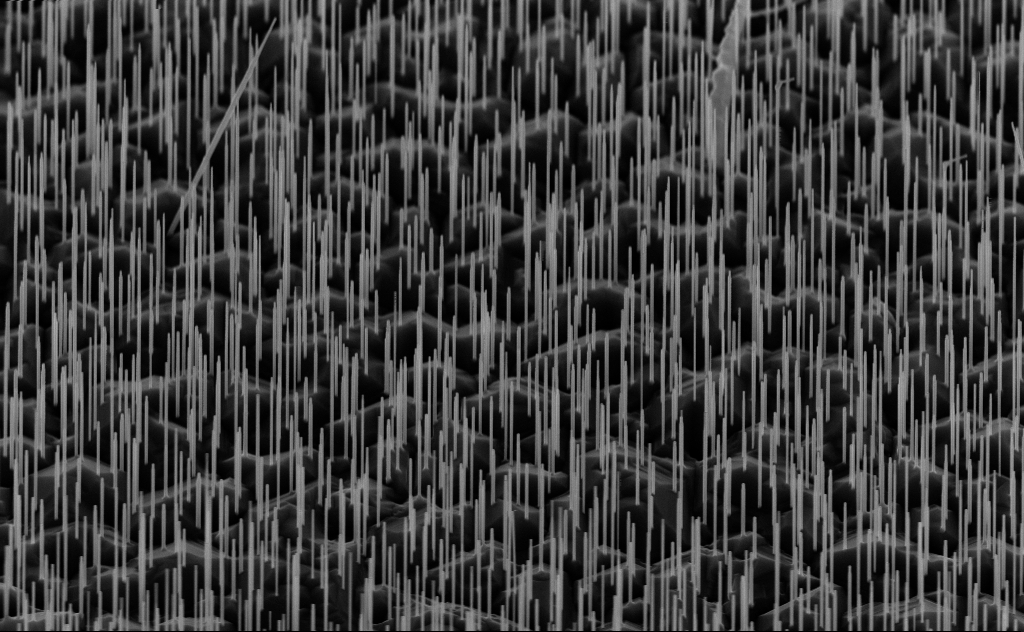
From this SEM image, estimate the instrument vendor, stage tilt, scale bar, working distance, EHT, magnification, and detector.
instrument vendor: Zeiss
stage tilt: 44.2°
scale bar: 2000 nm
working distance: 6 mm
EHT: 10 kV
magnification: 20 K X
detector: InLens